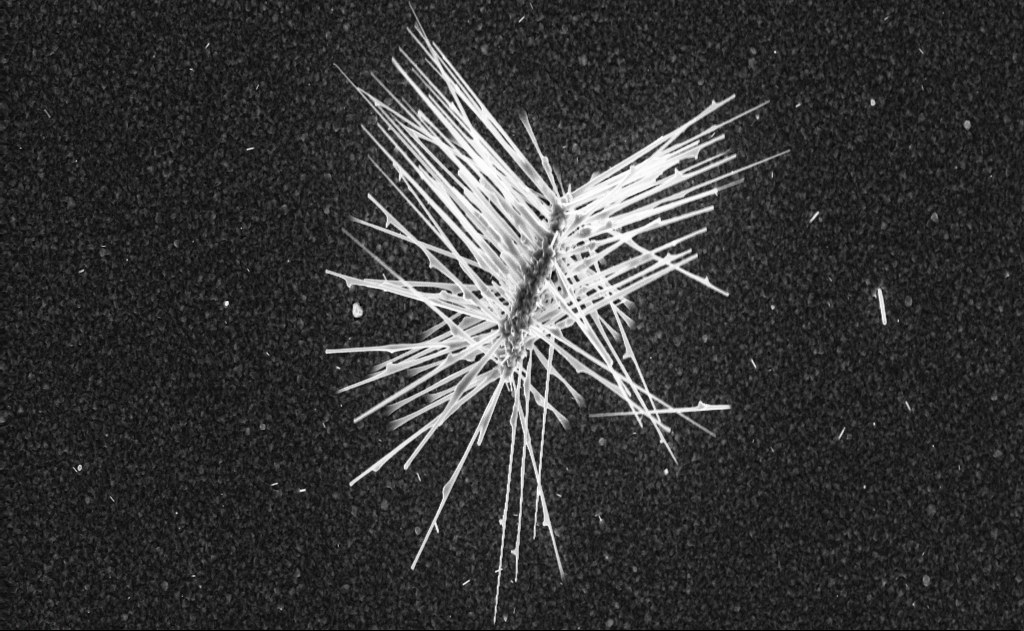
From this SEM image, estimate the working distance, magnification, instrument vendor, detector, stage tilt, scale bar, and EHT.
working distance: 6 mm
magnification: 4.55 K X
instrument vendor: Zeiss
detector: InLens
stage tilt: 0°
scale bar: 10000 nm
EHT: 10 kV